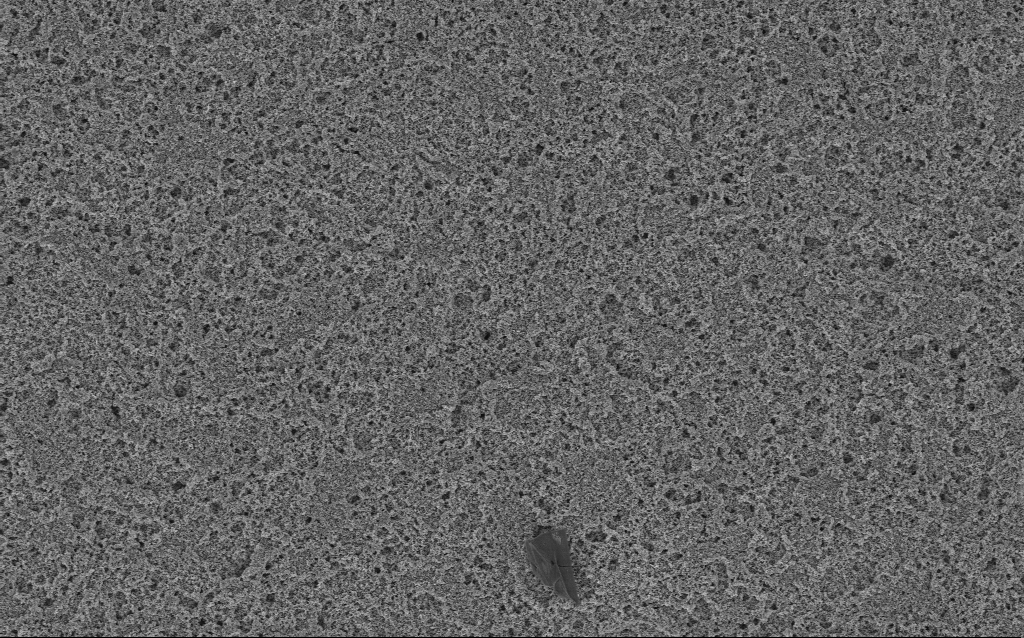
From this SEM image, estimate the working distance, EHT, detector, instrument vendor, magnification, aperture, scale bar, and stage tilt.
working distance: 10 mm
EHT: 3 kV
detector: InLens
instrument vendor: Zeiss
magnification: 6.61 K X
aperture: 30 µm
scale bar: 10000 nm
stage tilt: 0°